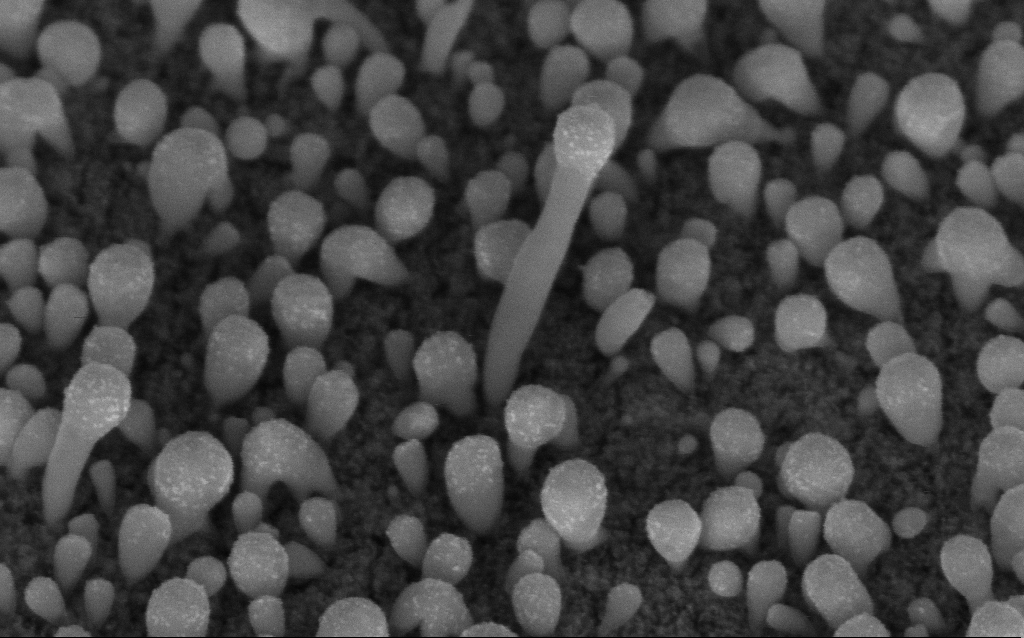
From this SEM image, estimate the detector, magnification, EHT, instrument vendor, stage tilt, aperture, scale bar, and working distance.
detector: InLens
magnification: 200 K X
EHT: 5 kV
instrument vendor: Zeiss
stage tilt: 45°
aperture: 30 µm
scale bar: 100 nm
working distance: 6.1 mm